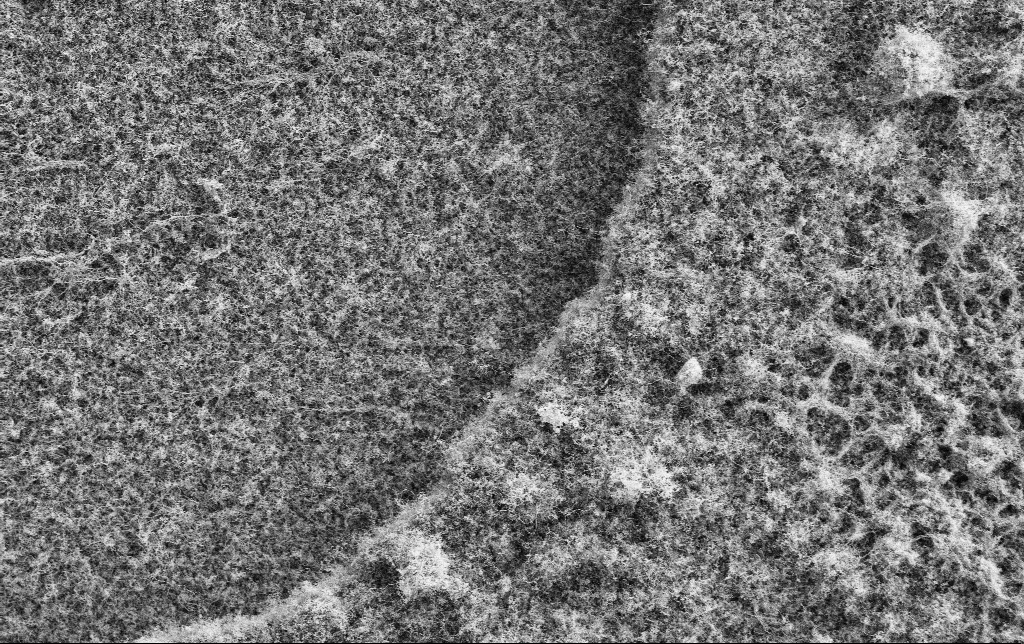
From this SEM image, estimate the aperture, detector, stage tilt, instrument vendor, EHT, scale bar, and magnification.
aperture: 30 µm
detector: SE2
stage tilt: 0°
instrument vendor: Zeiss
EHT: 2 kV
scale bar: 10000 nm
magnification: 2.5 K X